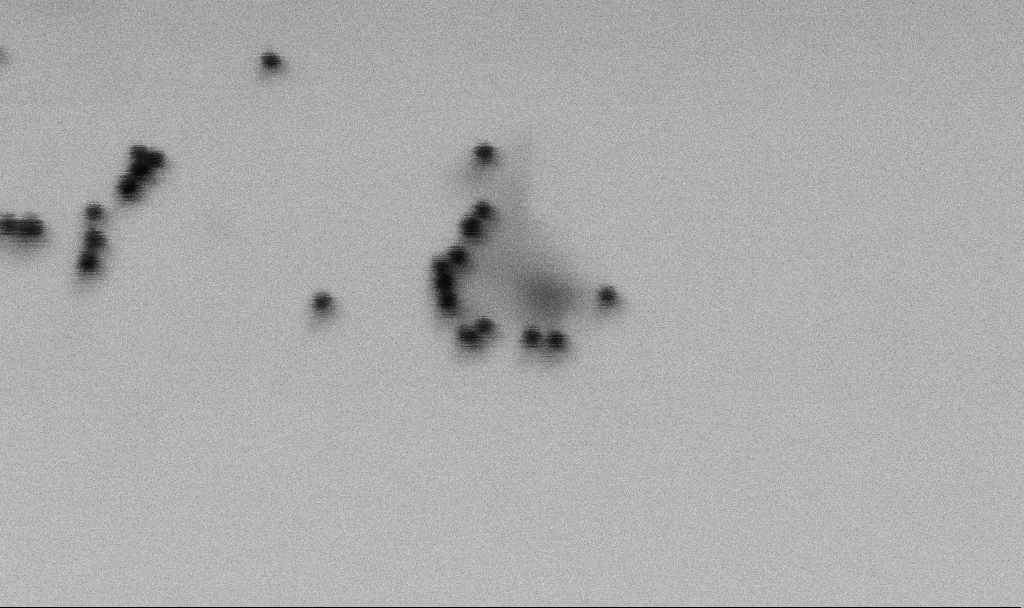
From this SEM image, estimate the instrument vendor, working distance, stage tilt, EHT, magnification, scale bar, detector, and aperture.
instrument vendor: Zeiss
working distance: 6.5 mm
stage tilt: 0°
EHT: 2 kV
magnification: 339.87 K X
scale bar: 200 nm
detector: SE2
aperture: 30 µm